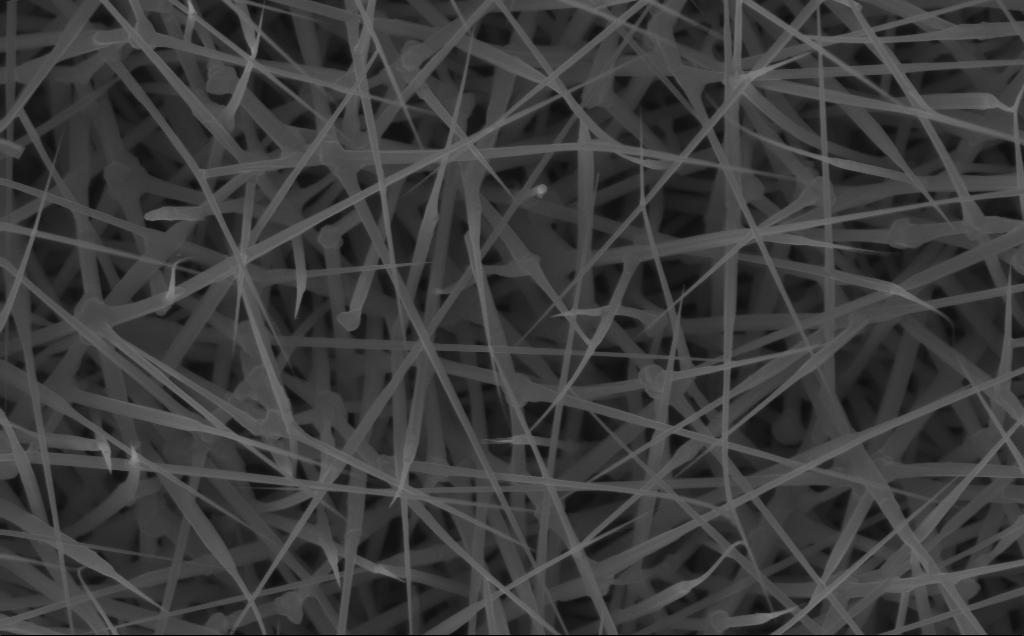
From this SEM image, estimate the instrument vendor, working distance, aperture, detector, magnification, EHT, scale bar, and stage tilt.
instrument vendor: Zeiss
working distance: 6 mm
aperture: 30 µm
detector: InLens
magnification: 40 K X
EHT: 10 kV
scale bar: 1000 nm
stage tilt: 0°